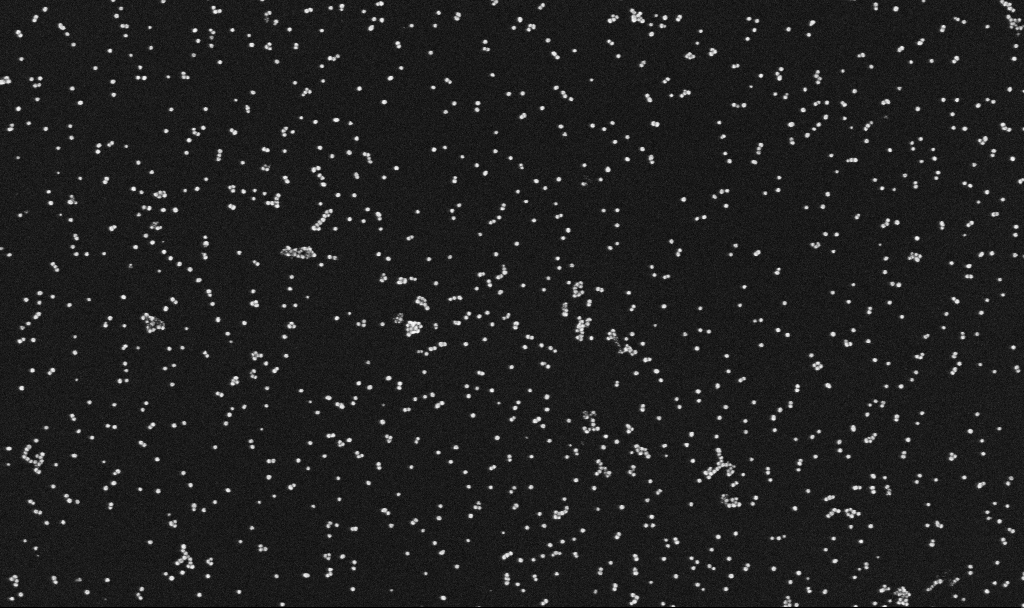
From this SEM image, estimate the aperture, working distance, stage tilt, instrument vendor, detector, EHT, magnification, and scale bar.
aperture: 30 µm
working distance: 3.4 mm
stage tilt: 0°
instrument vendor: Zeiss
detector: InLens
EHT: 10 kV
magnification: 100 K X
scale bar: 200 nm